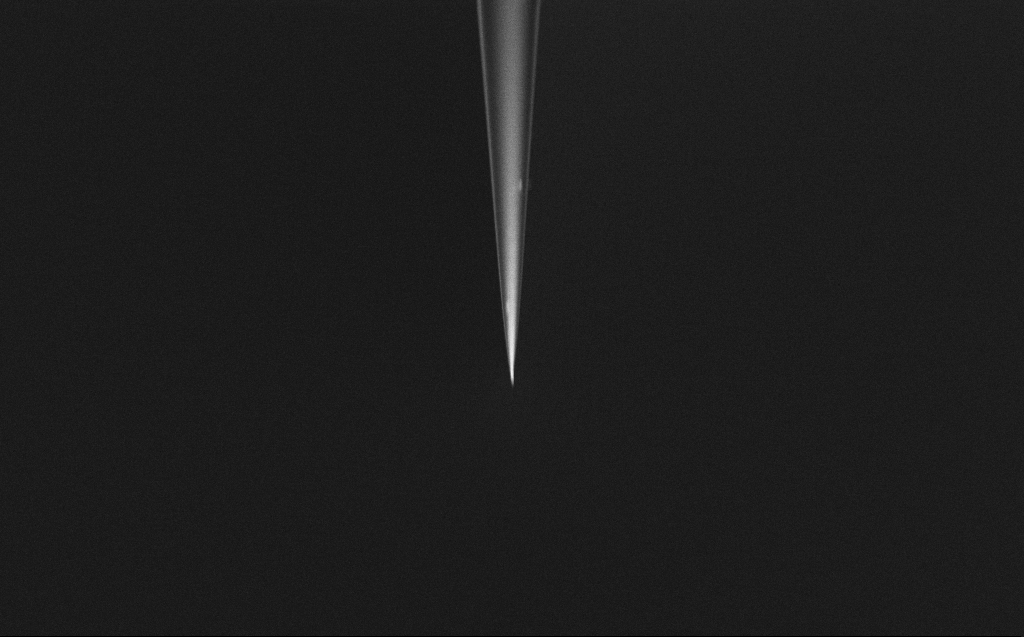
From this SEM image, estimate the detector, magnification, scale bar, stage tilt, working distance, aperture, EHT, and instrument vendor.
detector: InLens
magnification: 5 K X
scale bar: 10000 nm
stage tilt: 45°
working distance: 6 mm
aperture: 30 µm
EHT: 2 kV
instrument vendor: Zeiss